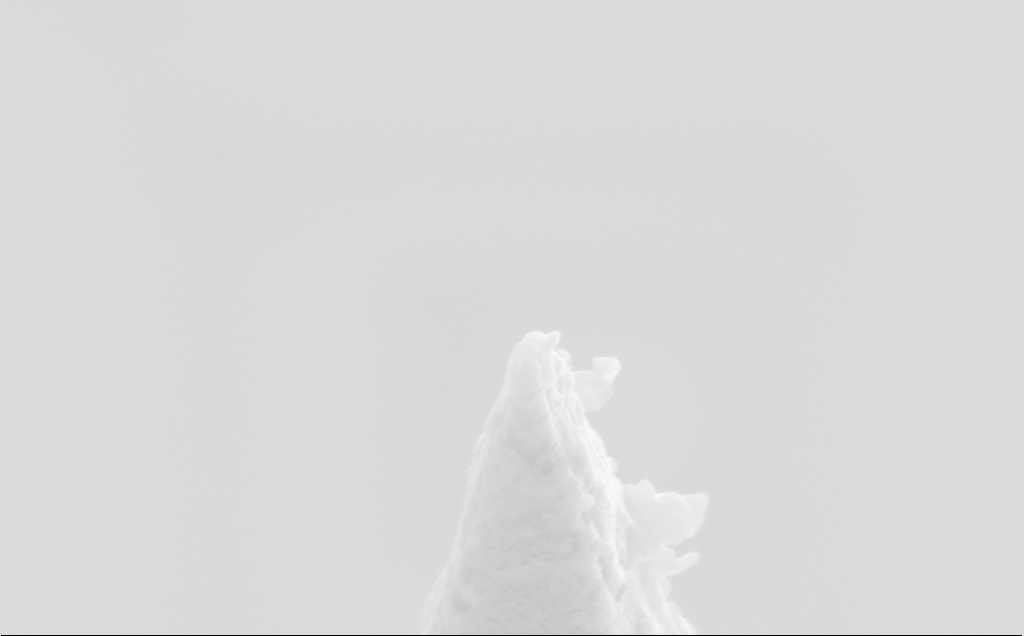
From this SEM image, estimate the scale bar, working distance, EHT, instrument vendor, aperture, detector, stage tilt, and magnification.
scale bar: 200 nm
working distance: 10 mm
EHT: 5 kV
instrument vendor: Zeiss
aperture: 30 µm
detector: SE2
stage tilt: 50°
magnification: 112.14 K X